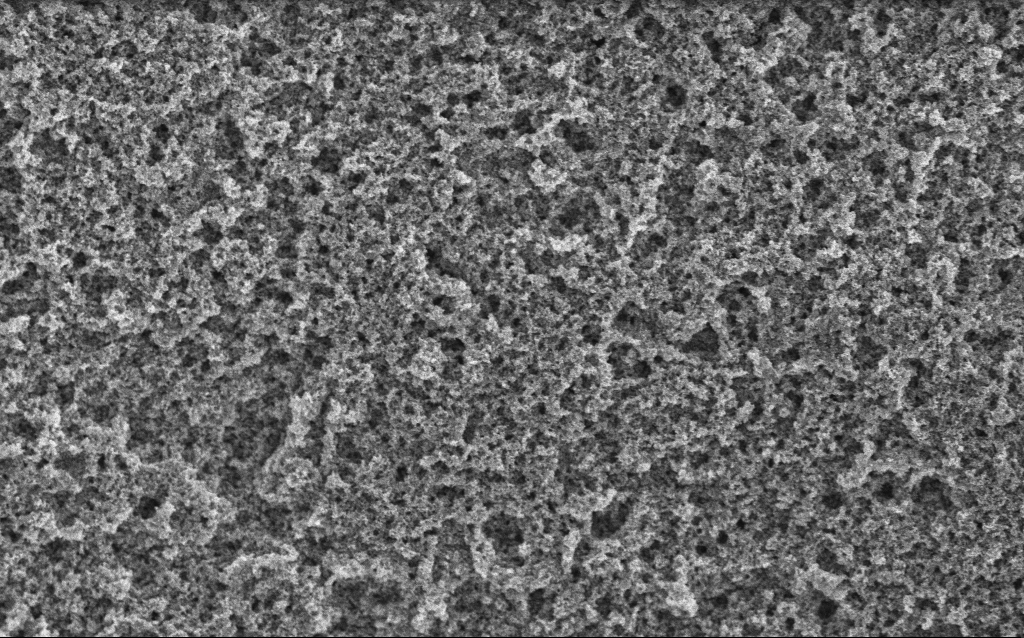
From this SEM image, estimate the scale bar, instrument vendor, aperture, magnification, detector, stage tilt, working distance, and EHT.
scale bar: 1000 nm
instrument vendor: Zeiss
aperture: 30 µm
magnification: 15.33 K X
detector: InLens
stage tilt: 0°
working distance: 4.4 mm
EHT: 5 kV